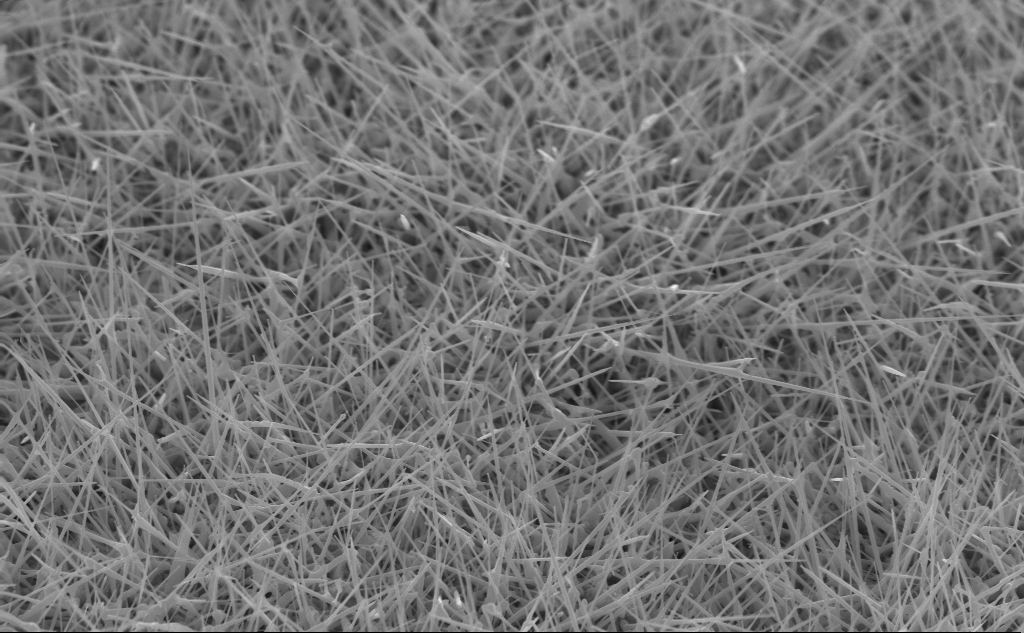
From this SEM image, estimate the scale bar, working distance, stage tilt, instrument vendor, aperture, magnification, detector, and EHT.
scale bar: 2000 nm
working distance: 4 mm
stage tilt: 45°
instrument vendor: Zeiss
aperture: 30 µm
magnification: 20 K X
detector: InLens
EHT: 10 kV